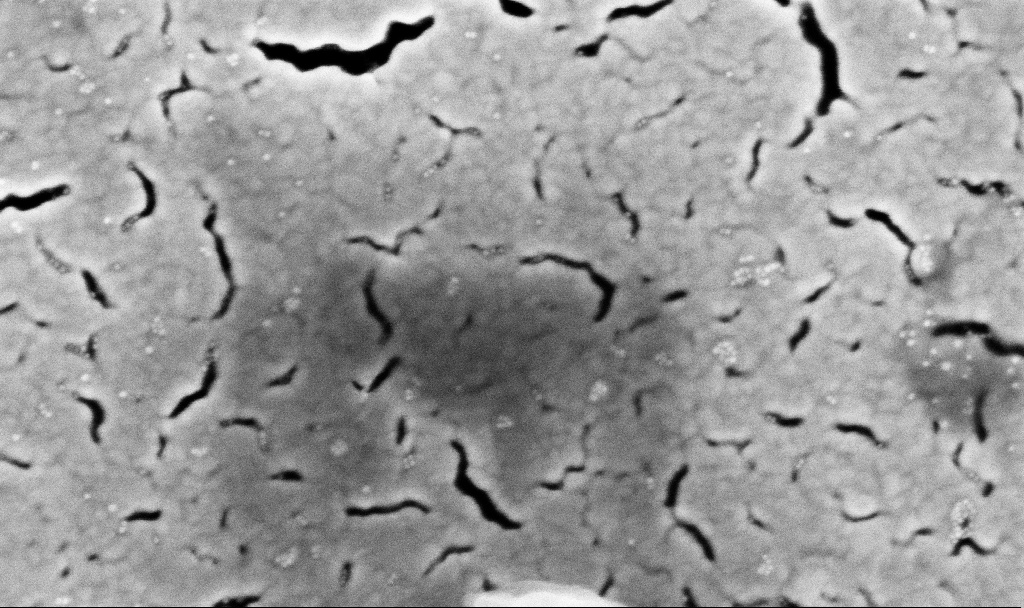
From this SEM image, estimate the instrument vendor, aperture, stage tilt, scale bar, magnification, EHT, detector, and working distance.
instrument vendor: Zeiss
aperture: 30 µm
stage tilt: -0.9°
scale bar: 100 nm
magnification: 164.75 K X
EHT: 5 kV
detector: InLens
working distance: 3.1 mm